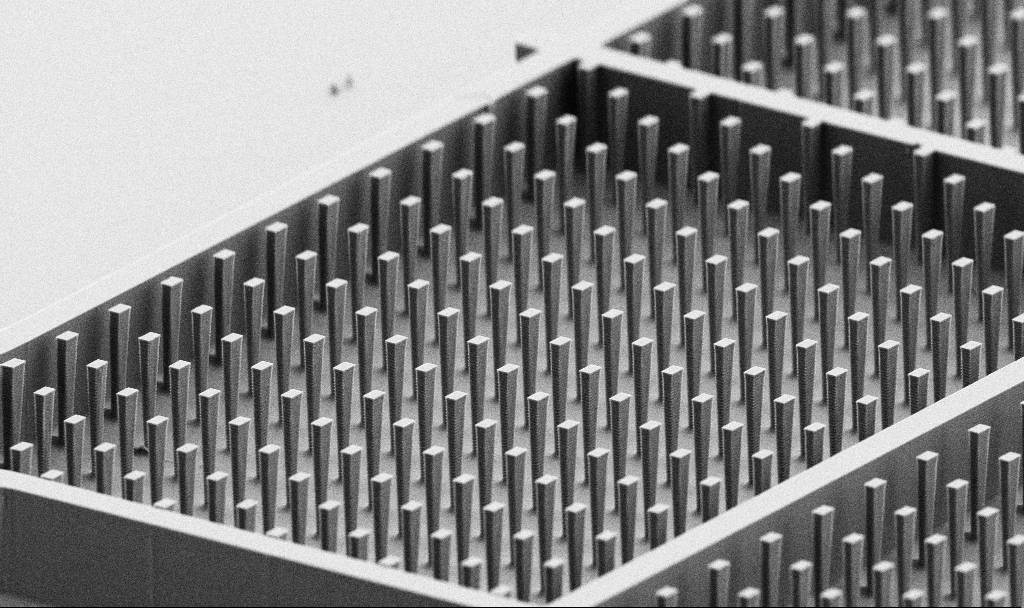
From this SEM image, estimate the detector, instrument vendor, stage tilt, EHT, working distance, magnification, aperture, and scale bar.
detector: SE2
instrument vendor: Zeiss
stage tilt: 70°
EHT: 5 kV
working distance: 6.3 mm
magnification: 2.8 K X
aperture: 30 µm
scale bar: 10000 nm